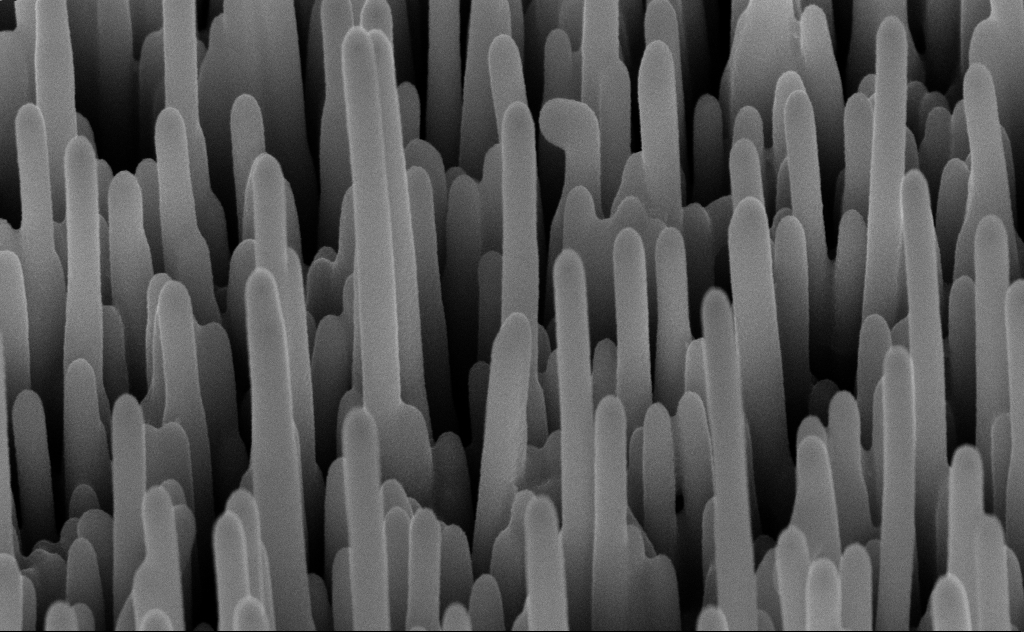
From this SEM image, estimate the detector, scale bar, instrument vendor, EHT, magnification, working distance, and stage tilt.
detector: InLens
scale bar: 200 nm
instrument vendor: Zeiss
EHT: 10 kV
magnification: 80 K X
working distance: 8 mm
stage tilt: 45°